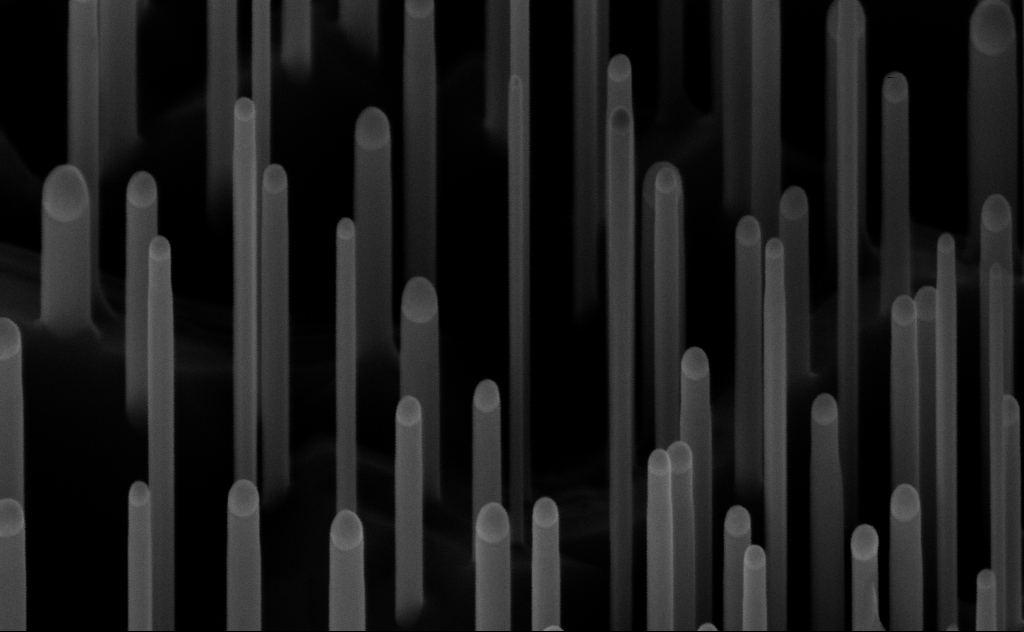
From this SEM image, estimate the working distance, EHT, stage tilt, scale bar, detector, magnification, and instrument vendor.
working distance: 7 mm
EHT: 10 kV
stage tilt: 45°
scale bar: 200 nm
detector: InLens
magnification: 150 K X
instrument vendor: Zeiss